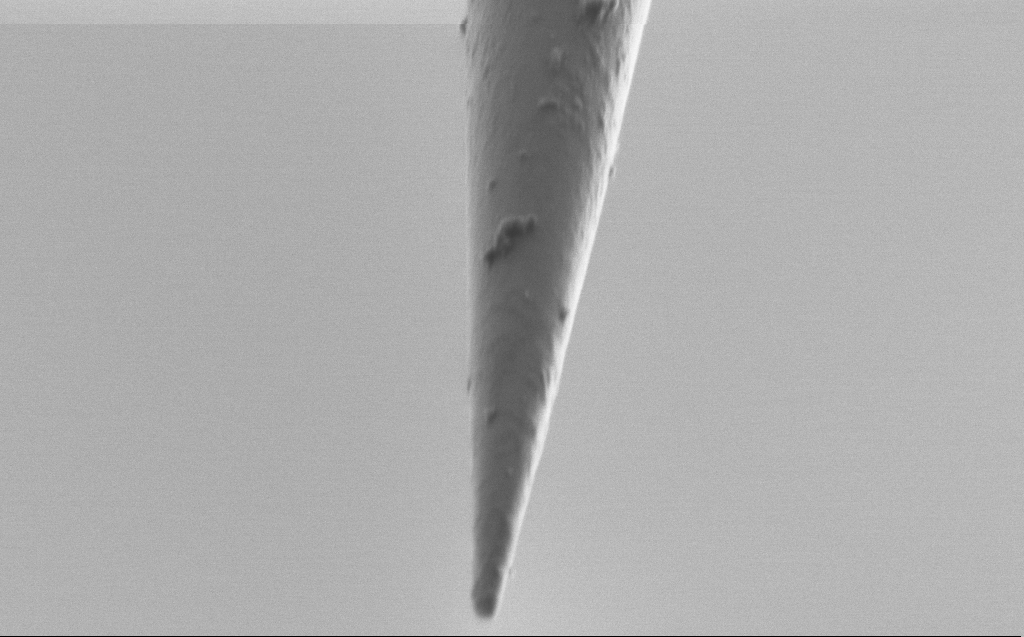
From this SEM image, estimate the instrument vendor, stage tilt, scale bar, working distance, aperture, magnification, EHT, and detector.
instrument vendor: Zeiss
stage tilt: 45°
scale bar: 1000 nm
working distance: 5 mm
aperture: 30 µm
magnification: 50 K X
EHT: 1 kV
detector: SE2